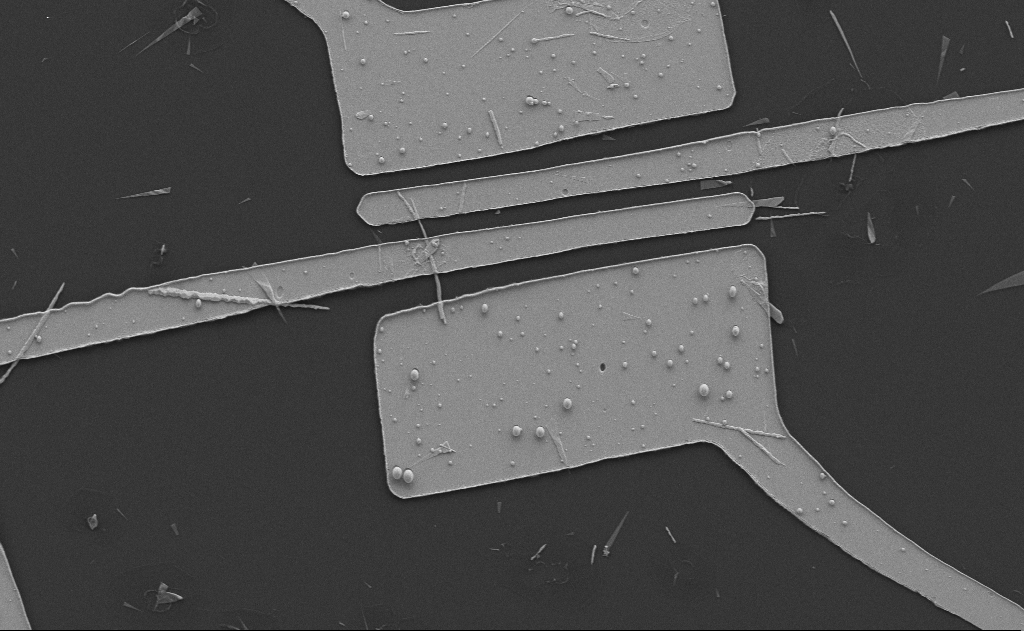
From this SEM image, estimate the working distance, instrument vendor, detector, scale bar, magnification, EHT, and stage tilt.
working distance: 10 mm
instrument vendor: Zeiss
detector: SE2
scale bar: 10000 nm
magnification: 4.94 K X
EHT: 5 kV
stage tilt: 0°